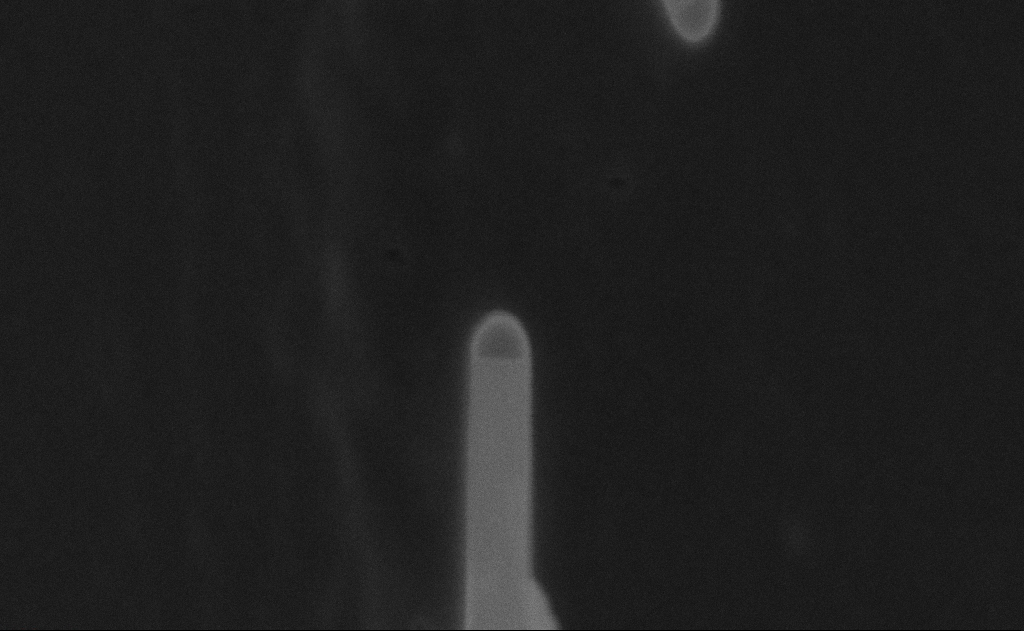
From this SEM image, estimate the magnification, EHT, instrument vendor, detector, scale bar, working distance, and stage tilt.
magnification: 352.32 K X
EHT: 27 kV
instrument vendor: Zeiss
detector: SE2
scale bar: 100 nm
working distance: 9 mm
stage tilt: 0°